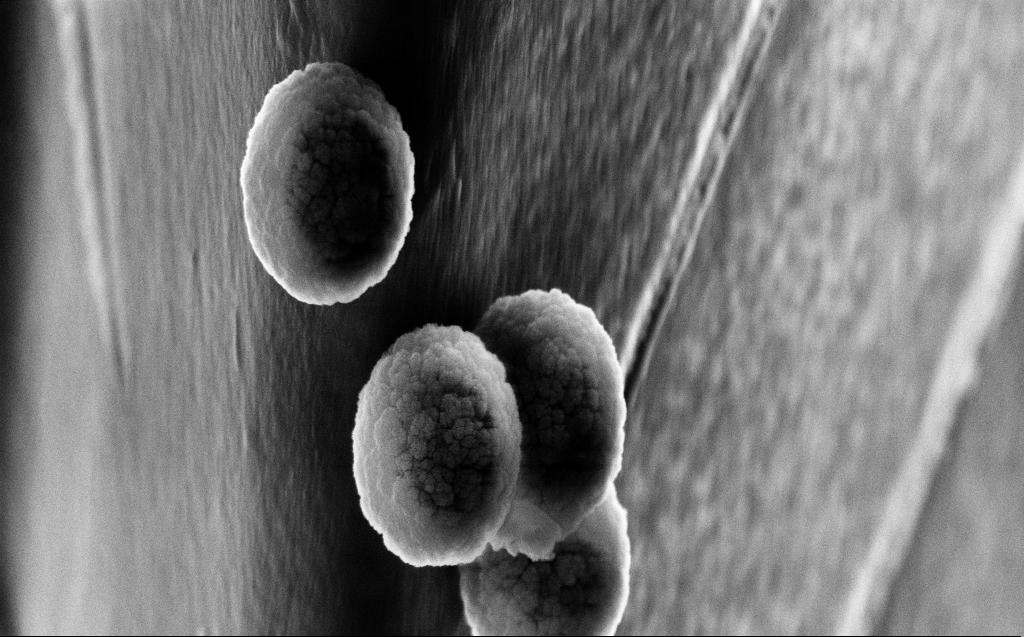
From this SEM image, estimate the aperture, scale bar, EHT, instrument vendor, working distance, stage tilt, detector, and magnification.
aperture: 30 µm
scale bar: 1000 nm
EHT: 10 kV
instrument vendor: Zeiss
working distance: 5 mm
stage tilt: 45°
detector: InLens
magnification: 42.99 K X